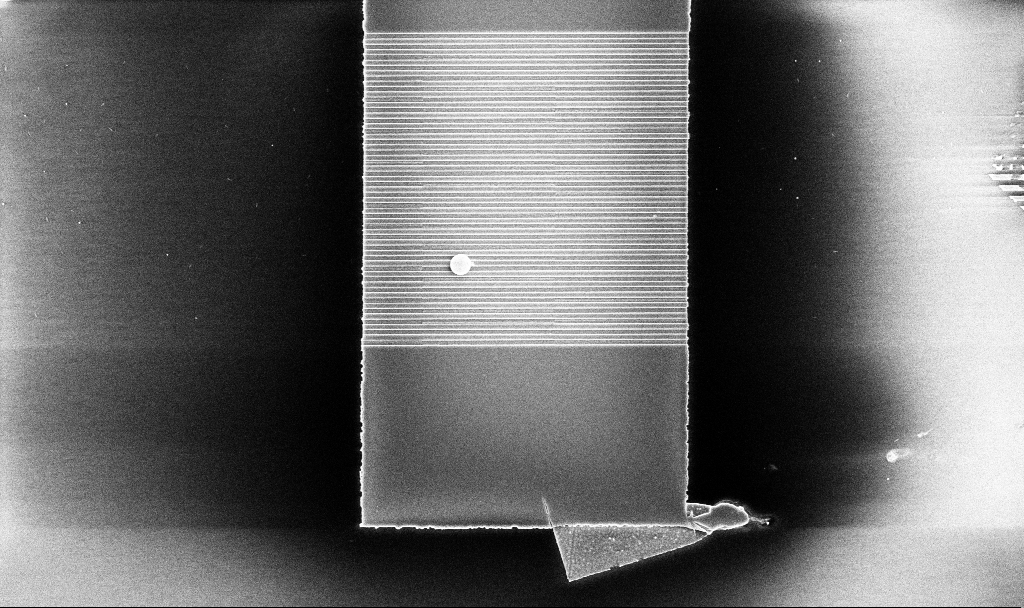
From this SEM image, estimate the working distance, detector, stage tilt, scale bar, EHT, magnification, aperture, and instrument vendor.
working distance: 10.1 mm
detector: InLens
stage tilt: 0°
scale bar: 10000 nm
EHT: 5 kV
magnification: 6 K X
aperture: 30 µm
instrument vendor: Zeiss